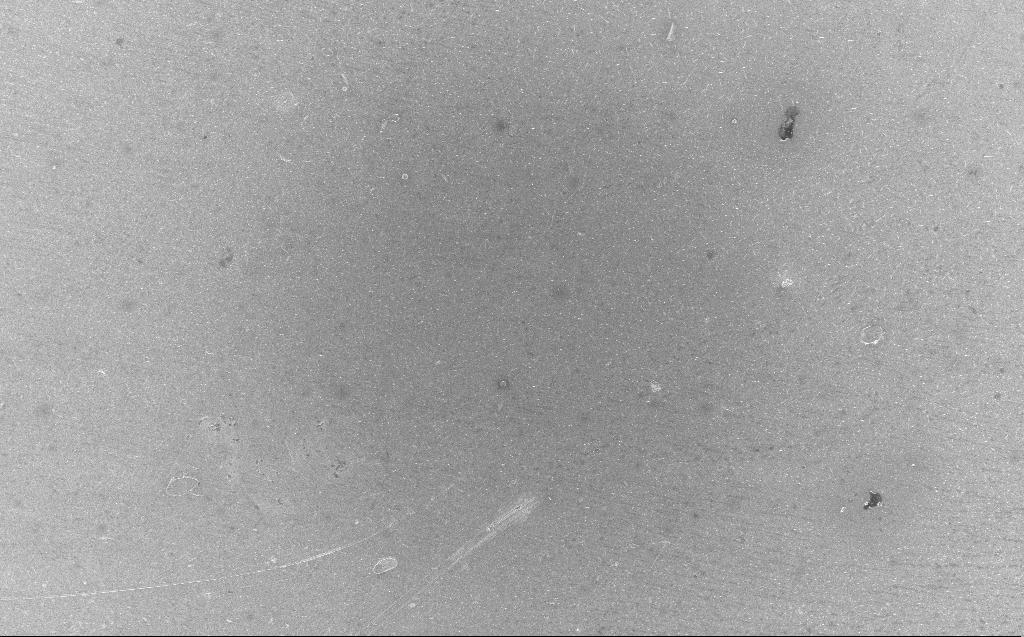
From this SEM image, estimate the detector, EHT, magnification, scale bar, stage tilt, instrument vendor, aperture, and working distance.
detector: InLens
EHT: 5 kV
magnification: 0.456 K X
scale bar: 100000 nm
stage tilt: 0°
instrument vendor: Zeiss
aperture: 30 µm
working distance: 4 mm